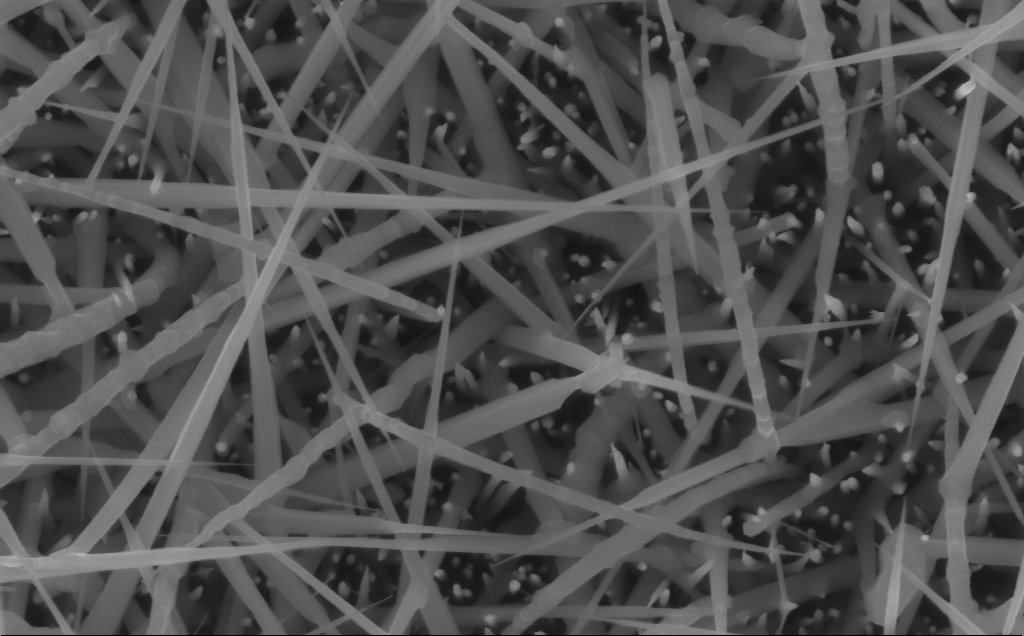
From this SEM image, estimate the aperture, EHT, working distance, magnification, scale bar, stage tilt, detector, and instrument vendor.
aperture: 30 µm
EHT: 10 kV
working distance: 7 mm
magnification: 80 K X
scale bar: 200 nm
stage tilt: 0°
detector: InLens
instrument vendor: Zeiss